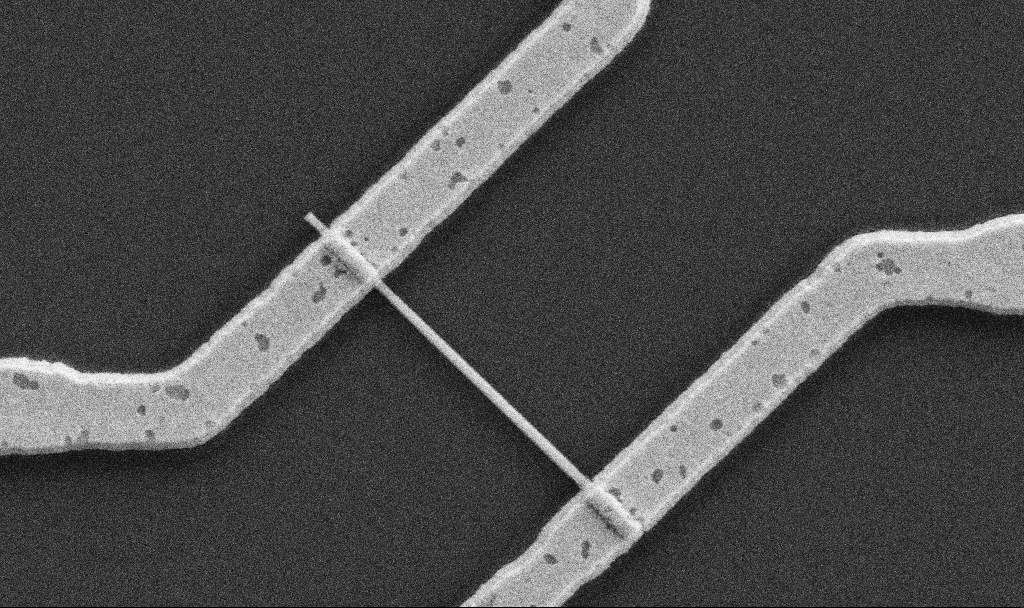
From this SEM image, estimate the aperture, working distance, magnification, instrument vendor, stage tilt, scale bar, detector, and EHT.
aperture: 30 µm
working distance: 10.7 mm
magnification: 42.43 K X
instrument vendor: Zeiss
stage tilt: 0°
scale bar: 1000 nm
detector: SE2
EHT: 5 kV